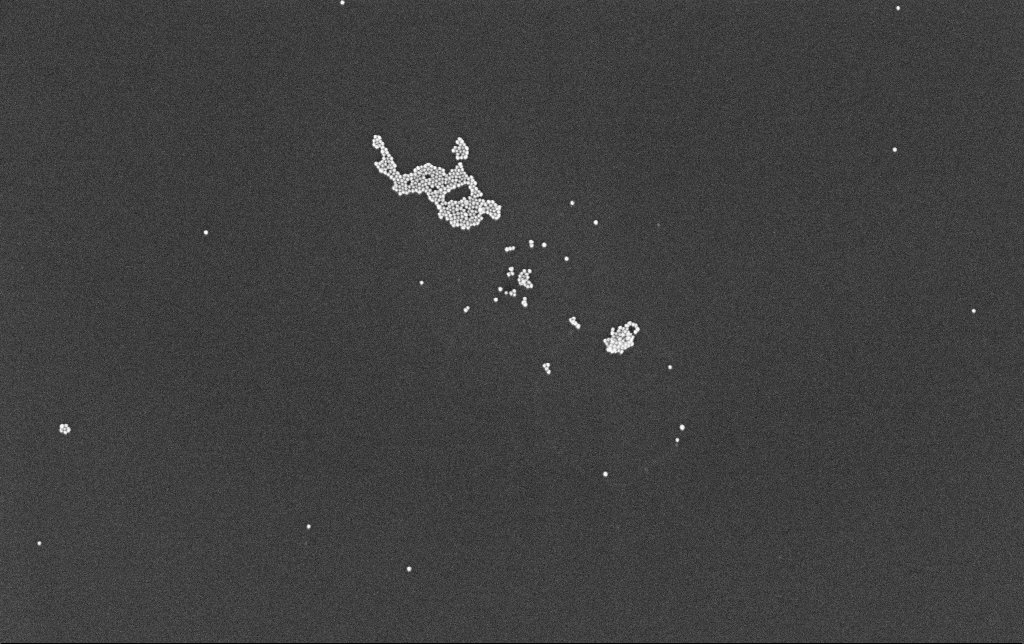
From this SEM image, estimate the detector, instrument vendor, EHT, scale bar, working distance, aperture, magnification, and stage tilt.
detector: InLens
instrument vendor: Zeiss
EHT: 10 kV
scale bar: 200 nm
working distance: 3.4 mm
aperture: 30 µm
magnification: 100 K X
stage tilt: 0°